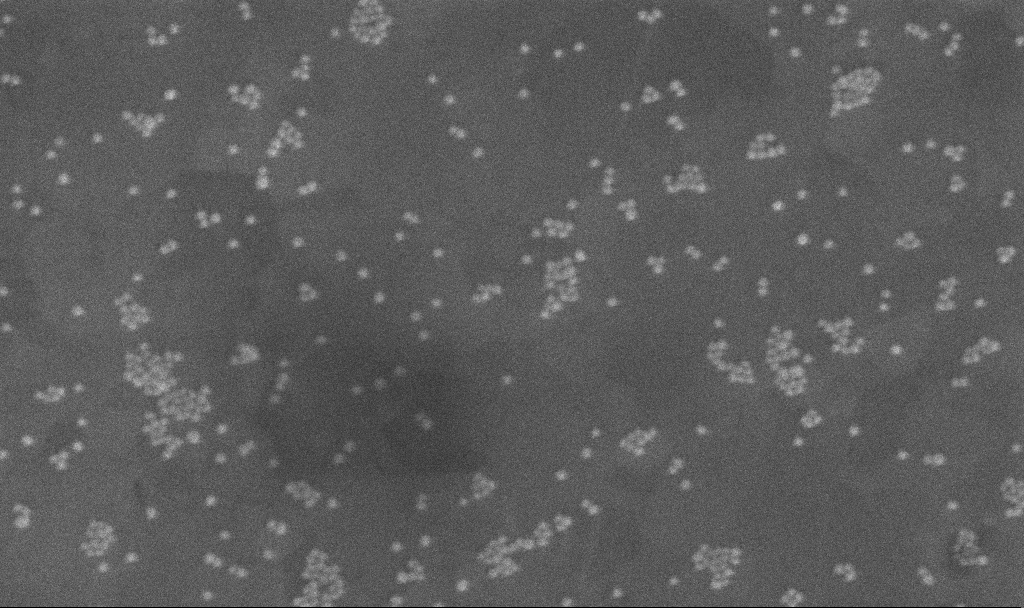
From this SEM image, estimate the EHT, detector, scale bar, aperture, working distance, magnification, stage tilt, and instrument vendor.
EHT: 10 kV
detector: InLens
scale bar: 200 nm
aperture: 30 µm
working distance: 3.9 mm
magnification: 198.62 K X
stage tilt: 0°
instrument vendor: Zeiss